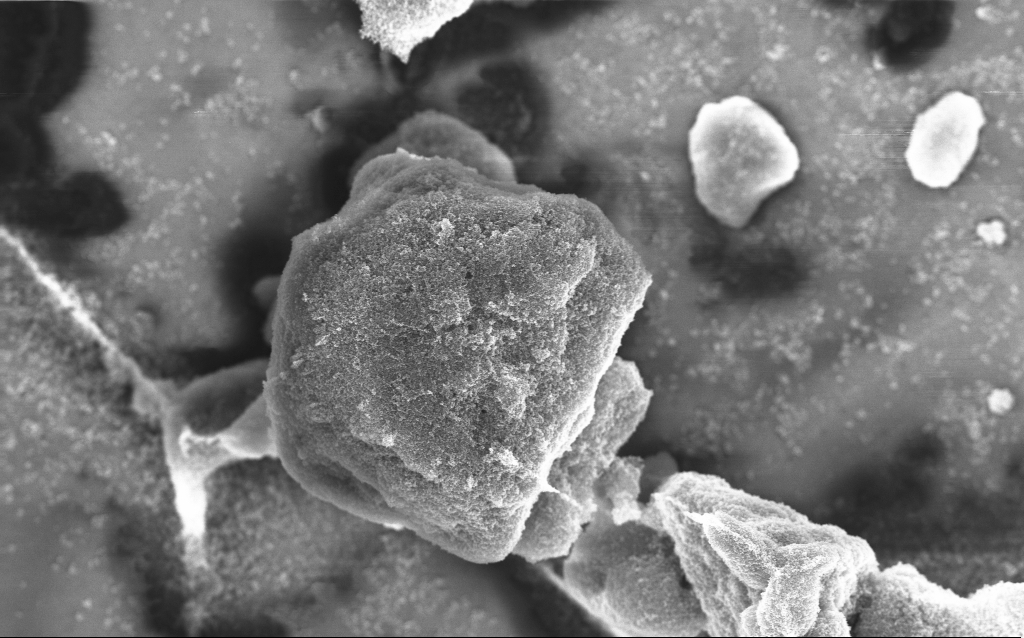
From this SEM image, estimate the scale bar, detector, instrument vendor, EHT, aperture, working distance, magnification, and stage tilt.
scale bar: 1000 nm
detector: InLens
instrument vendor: Zeiss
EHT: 10 kV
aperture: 30 µm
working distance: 2.8 mm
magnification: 15.33 K X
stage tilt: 0°